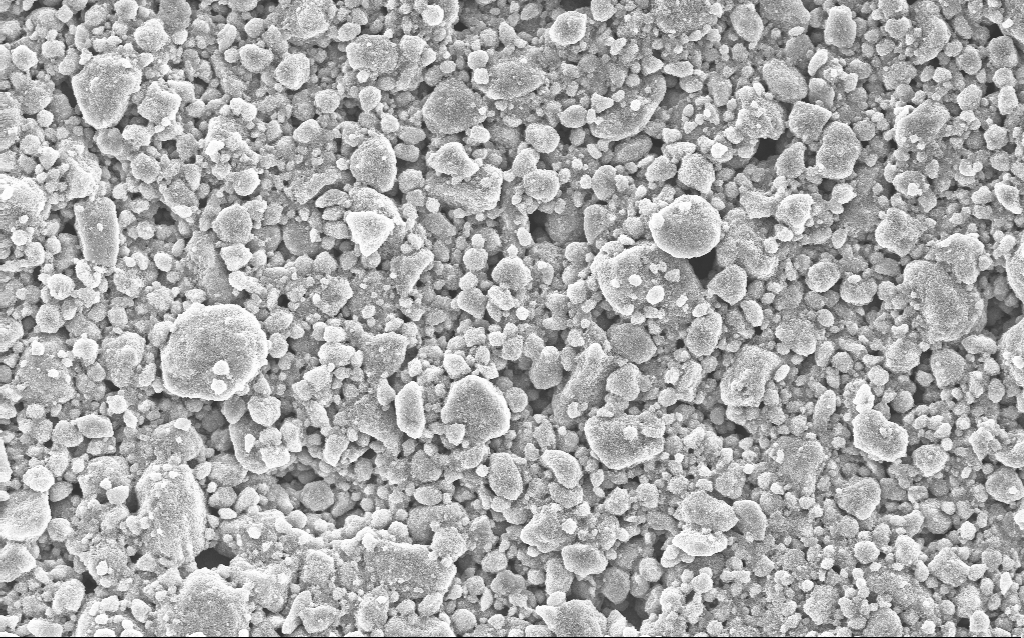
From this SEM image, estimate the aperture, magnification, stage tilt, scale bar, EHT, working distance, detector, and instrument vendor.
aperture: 30 µm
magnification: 3.39 K X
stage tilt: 0°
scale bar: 10000 nm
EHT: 10 kV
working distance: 3.6 mm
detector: InLens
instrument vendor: Zeiss